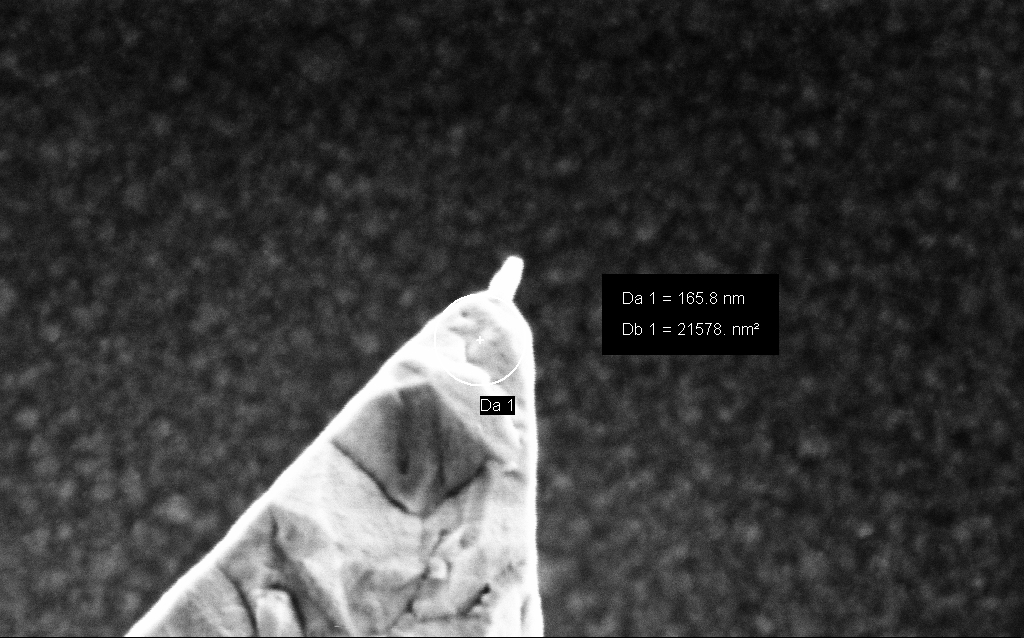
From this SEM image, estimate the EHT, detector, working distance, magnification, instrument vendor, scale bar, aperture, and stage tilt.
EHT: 3 kV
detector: InLens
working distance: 4.5 mm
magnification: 203.8 K X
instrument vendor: Zeiss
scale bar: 200 nm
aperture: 30 µm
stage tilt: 0°